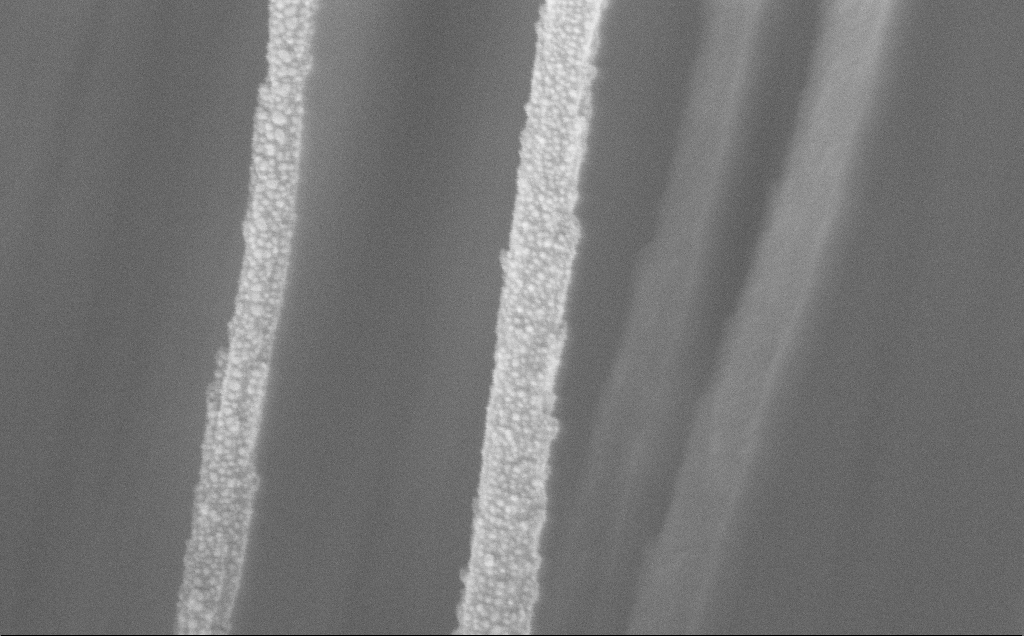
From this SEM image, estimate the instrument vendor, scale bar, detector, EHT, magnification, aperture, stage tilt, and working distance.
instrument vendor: Zeiss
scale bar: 200 nm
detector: InLens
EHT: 5 kV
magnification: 163.96 K X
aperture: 30 µm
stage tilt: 0°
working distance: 11 mm